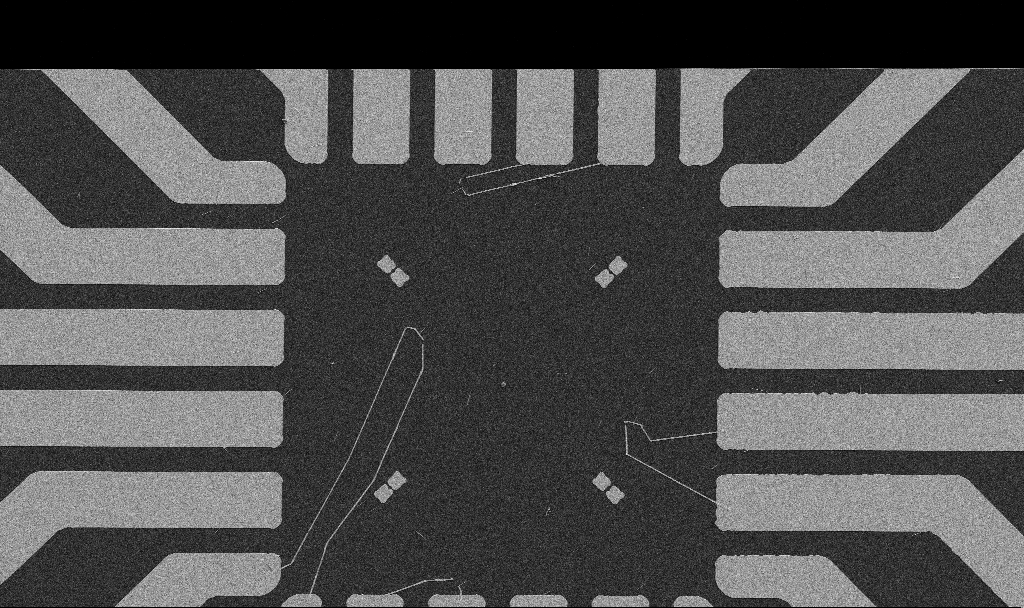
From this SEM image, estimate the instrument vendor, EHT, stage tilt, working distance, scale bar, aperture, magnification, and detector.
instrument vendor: Zeiss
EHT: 5 kV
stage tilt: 0°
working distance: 10.7 mm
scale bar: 20000 nm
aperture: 30 µm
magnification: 1 K X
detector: SE2